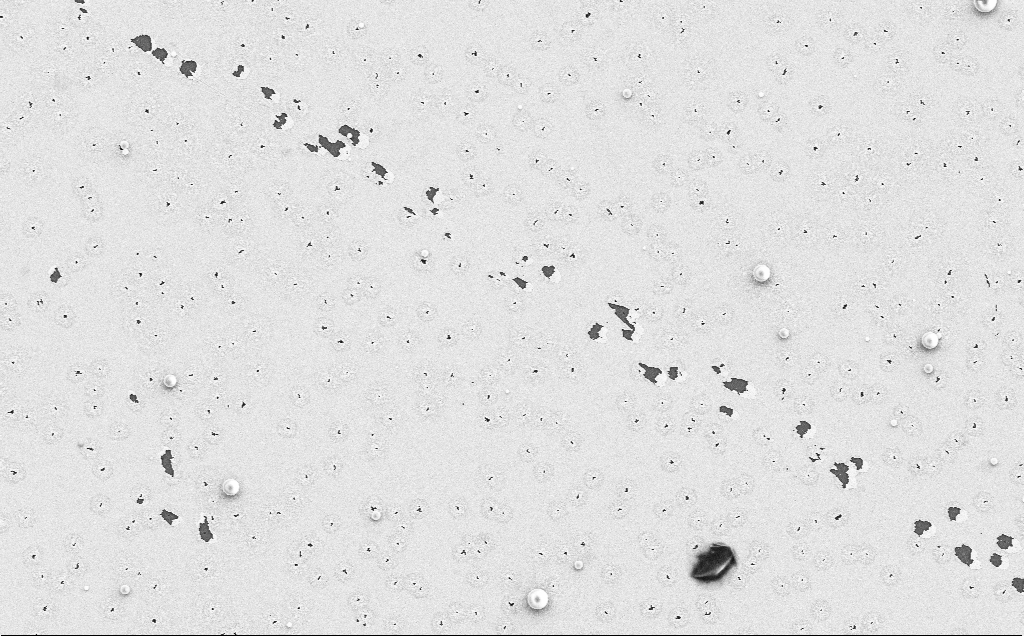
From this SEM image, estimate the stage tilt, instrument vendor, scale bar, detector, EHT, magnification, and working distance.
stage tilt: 0°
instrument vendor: Zeiss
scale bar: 10000 nm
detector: SE2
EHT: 5 kV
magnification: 4.2 K X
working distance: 12 mm